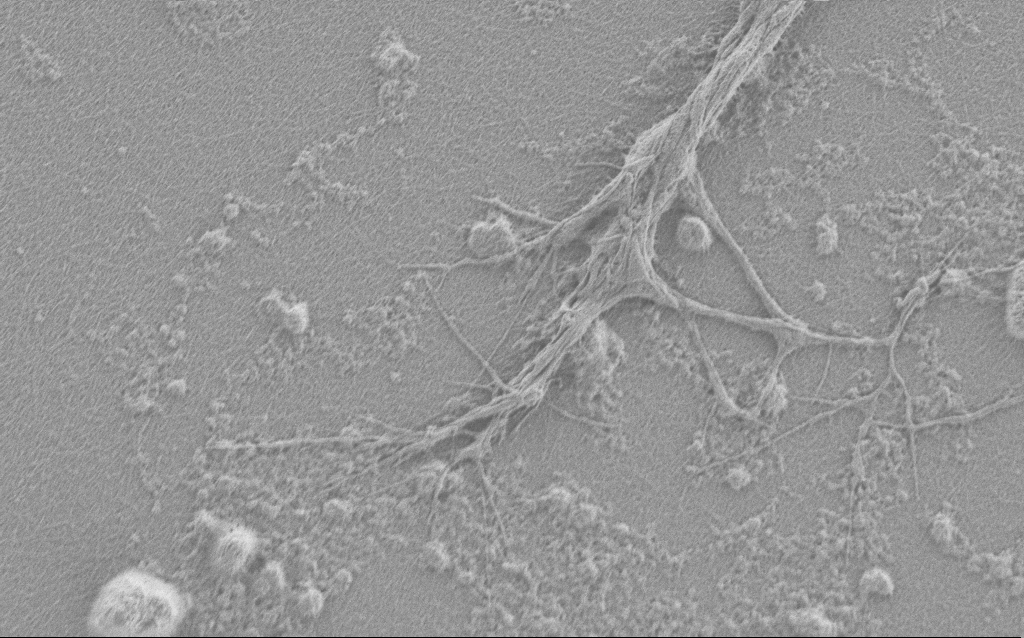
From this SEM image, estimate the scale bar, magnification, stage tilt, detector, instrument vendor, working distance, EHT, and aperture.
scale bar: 2000 nm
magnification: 7.5 K X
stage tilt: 0°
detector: SE2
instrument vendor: Zeiss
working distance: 4 mm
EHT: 0.9 kV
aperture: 30 µm